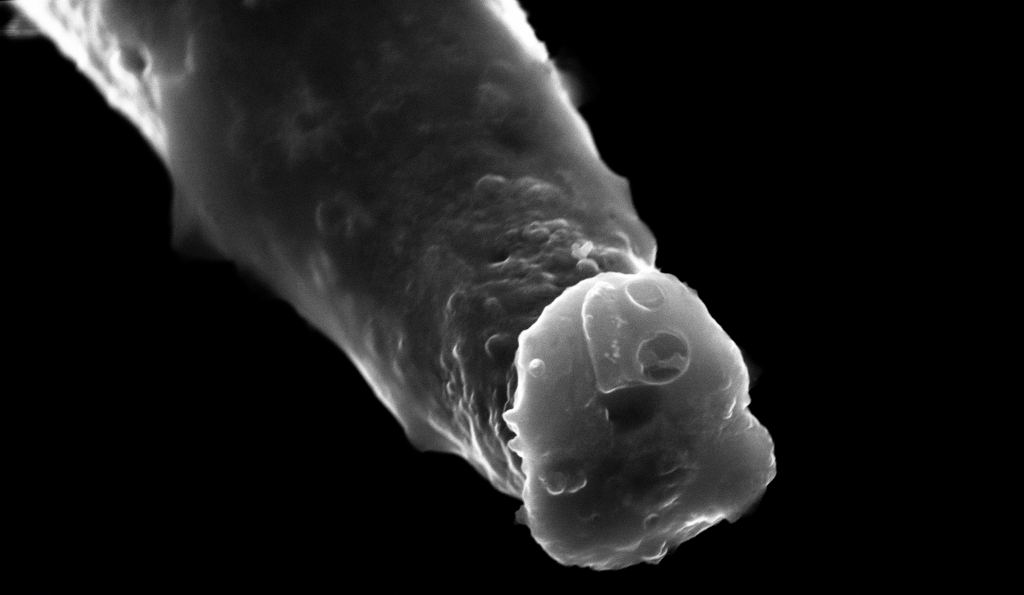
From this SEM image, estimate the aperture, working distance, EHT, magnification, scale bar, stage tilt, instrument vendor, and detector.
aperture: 30 µm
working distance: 2.2 mm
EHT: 10 kV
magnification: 60.58 K X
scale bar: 1000 nm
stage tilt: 56°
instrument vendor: Zeiss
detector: InLens